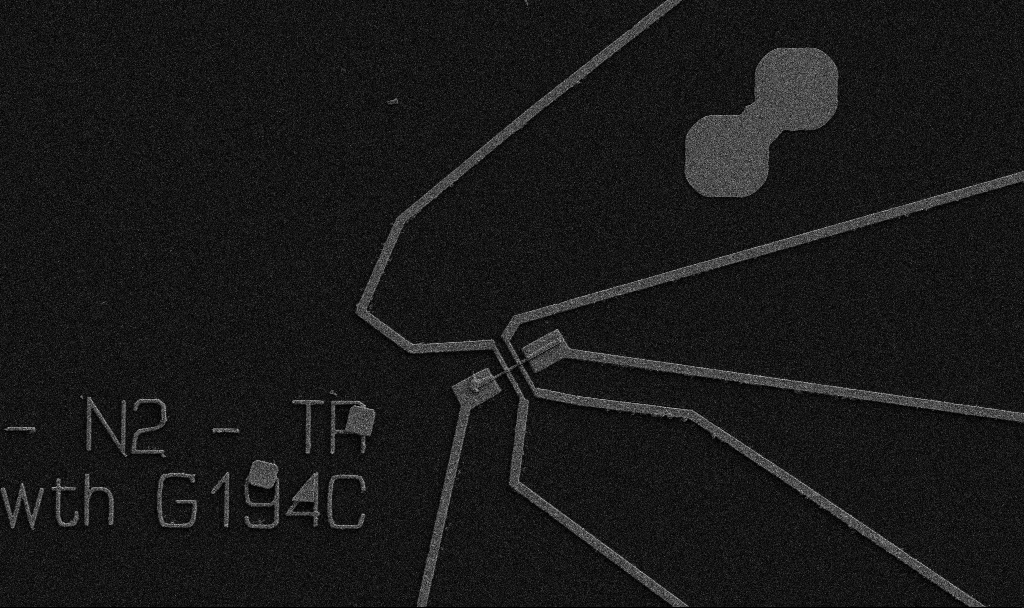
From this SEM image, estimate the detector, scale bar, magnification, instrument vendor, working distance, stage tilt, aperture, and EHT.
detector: SE2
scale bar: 10000 nm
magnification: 5 K X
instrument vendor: Zeiss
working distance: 10.7 mm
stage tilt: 0°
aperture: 30 µm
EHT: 5 kV